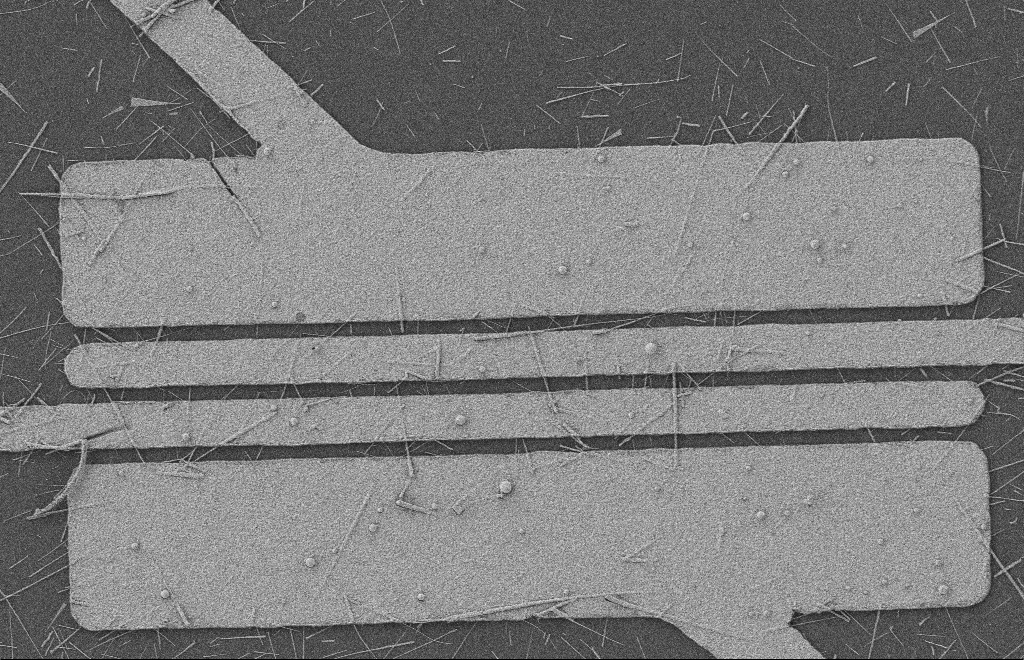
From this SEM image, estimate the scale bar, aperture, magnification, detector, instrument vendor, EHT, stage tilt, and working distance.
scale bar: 2000 nm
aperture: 20 µm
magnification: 5.52 K X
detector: SE2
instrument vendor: Zeiss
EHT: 2 kV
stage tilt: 0°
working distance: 8 mm